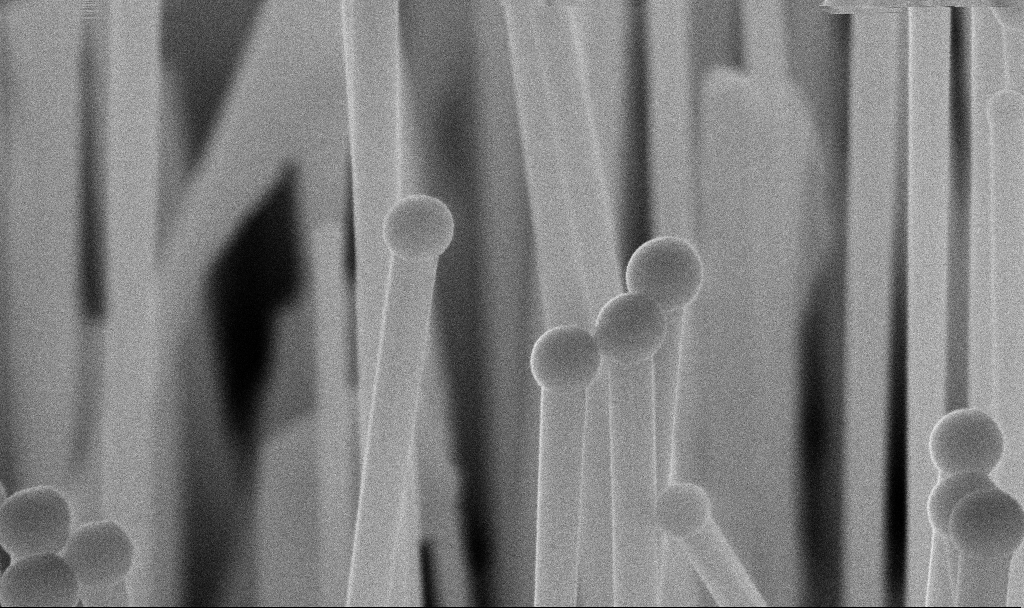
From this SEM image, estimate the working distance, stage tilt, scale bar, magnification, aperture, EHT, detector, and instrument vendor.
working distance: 7.2 mm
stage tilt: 45°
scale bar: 200 nm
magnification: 142.89 K X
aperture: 30 µm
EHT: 10 kV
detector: InLens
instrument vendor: Zeiss